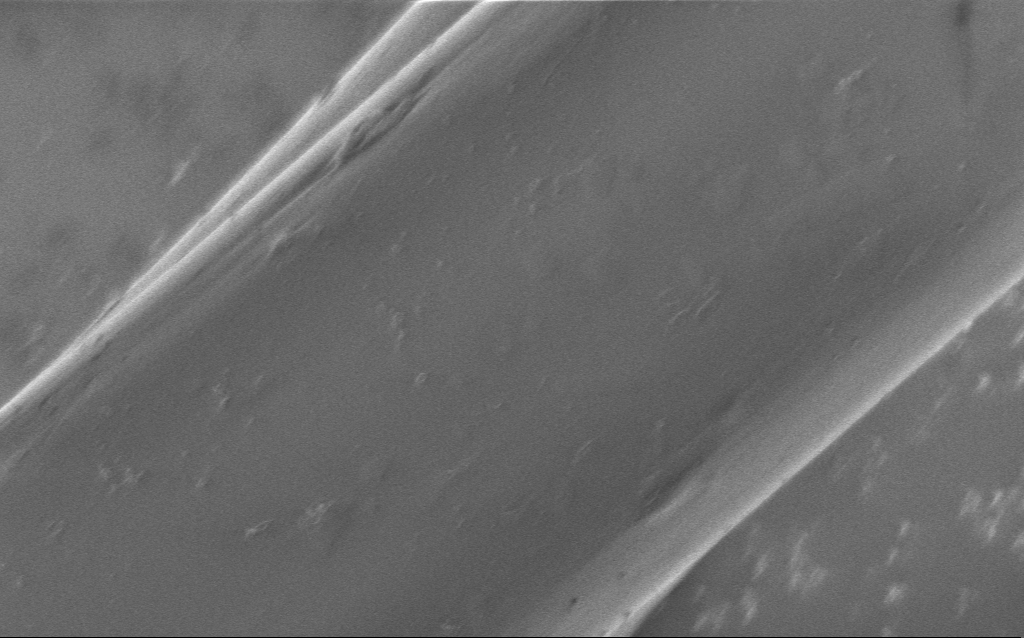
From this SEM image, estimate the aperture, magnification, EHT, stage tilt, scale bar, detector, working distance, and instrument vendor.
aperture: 30 µm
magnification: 16.4 K X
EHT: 1 kV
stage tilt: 0°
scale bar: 1000 nm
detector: InLens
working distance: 5 mm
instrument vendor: Zeiss